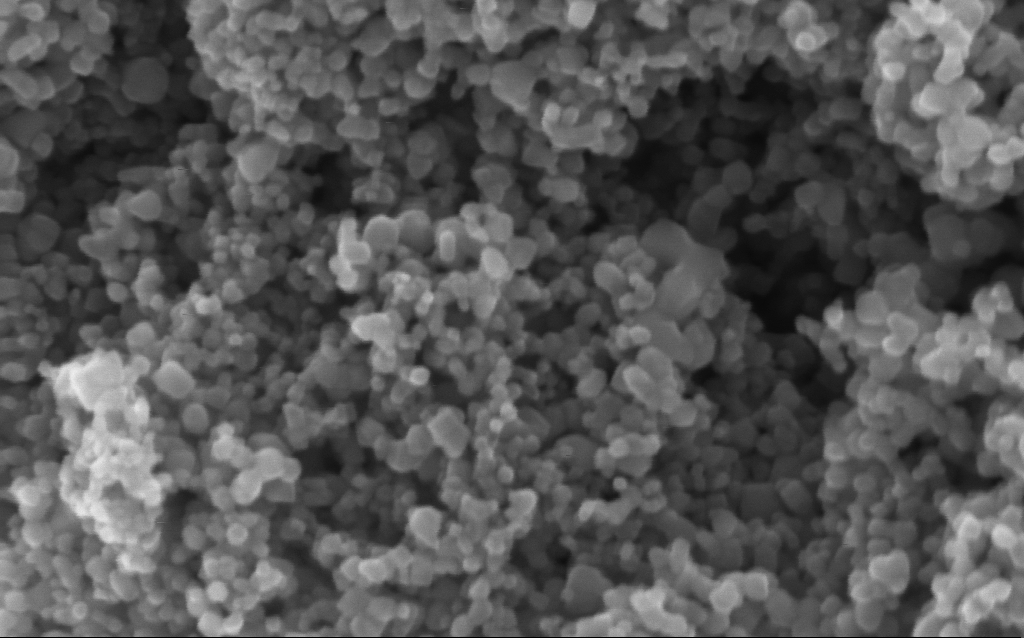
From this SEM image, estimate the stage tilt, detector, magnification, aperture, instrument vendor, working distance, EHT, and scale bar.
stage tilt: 0°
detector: InLens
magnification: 294.05 K X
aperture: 30 µm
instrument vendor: Zeiss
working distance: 4.2 mm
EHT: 5 kV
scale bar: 200 nm